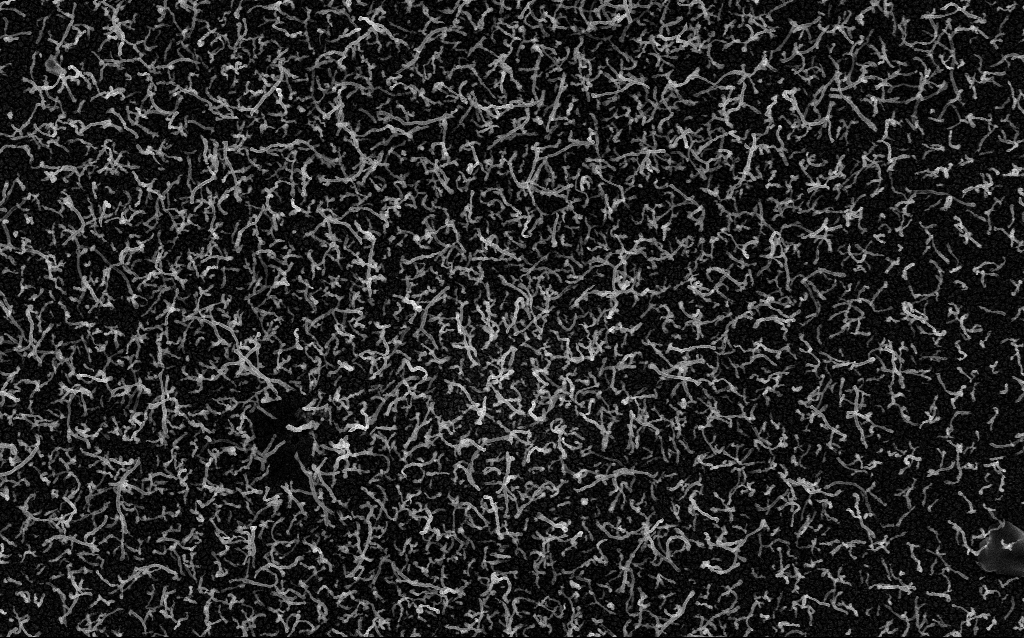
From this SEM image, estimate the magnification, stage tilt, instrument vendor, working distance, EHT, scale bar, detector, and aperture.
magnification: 20 K X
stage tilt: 0°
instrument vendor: Zeiss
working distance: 2.2 mm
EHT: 5 kV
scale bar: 2000 nm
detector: InLens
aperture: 30 µm